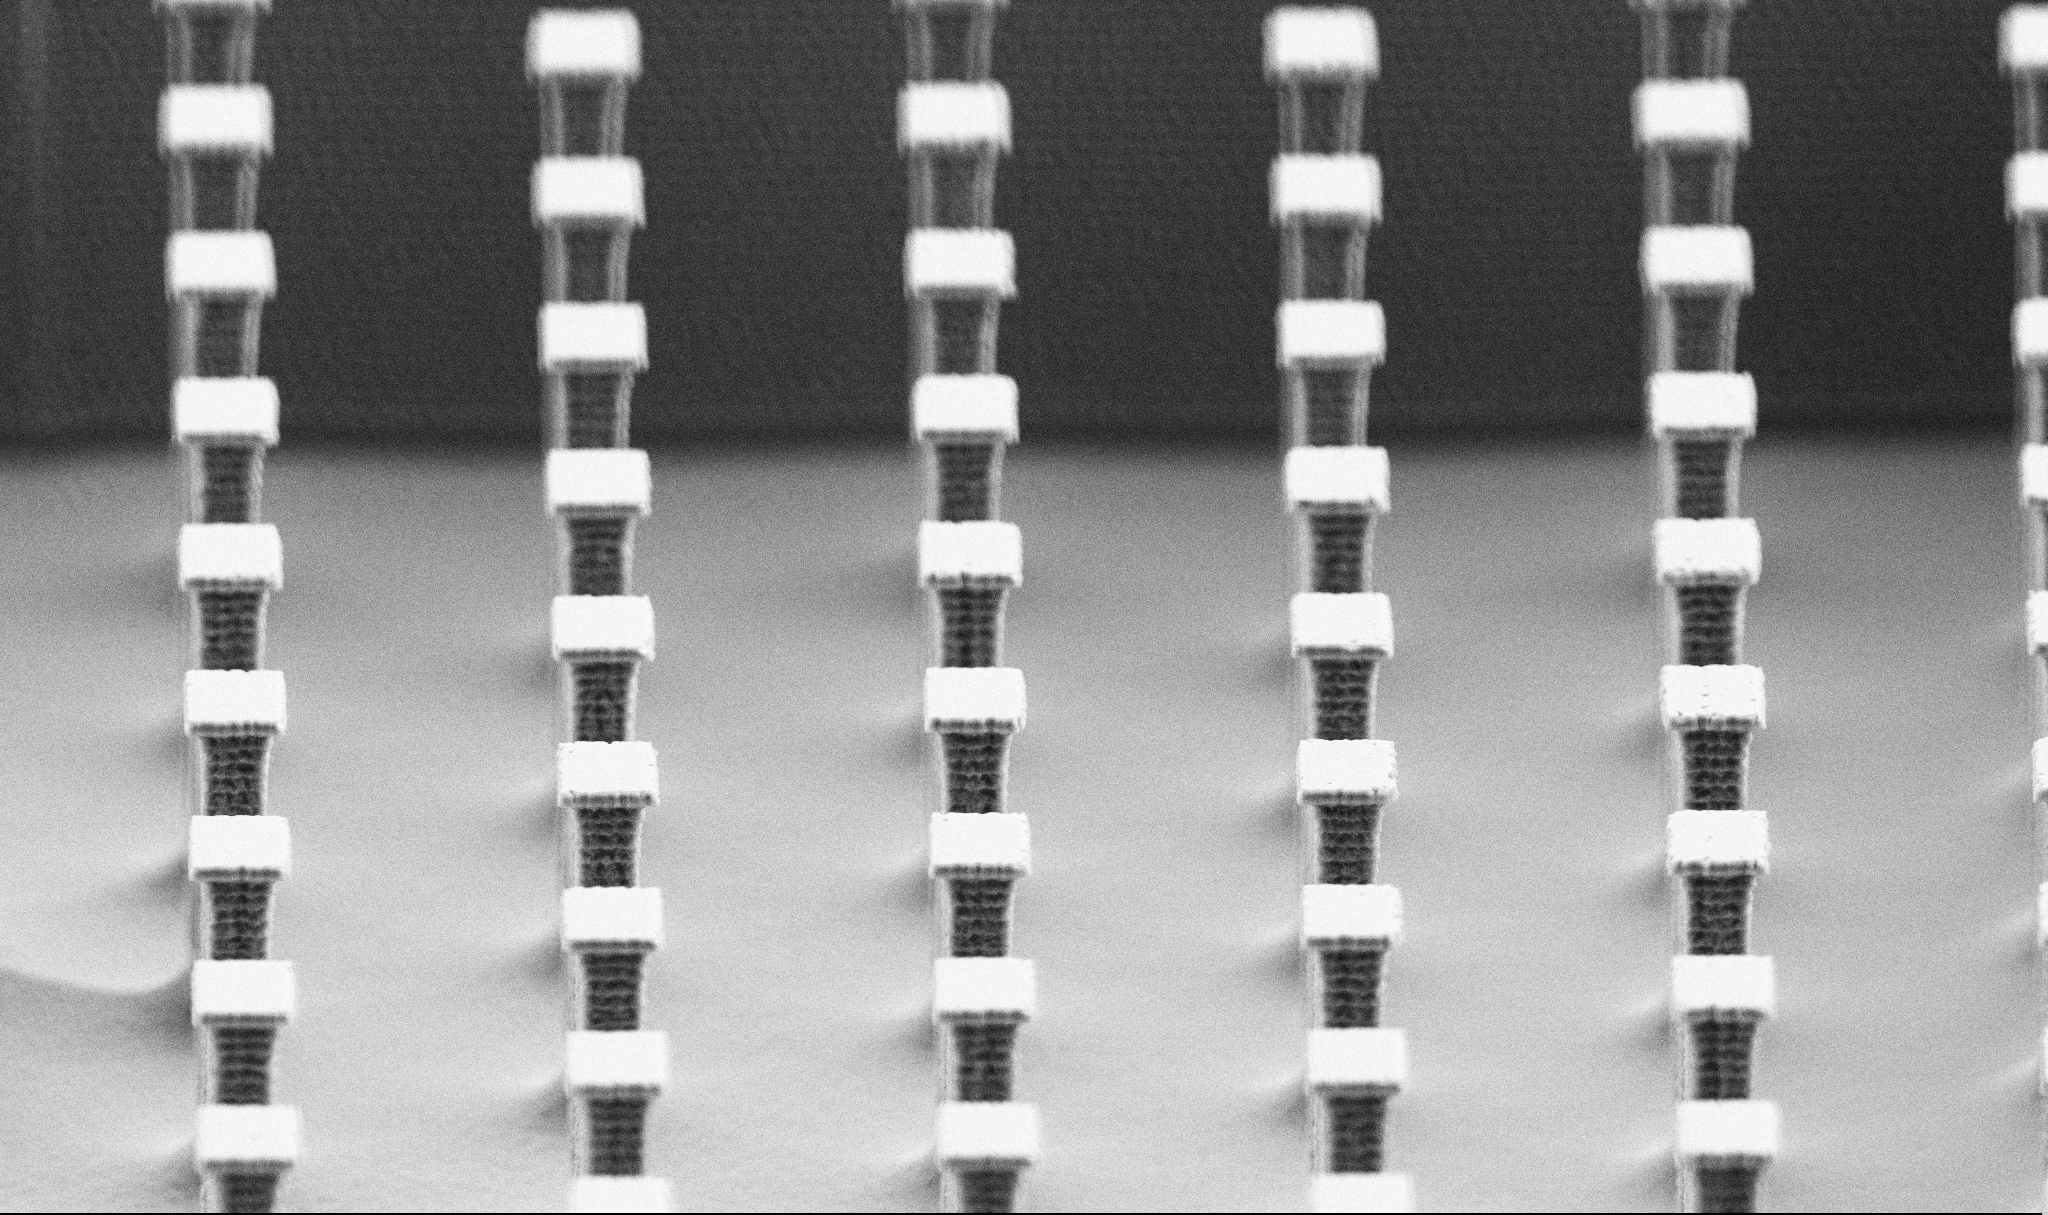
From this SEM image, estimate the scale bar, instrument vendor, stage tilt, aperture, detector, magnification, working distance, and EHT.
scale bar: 10000 nm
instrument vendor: Zeiss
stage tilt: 70°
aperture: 30 µm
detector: SE2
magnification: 6.53 K X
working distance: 6 mm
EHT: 5 kV